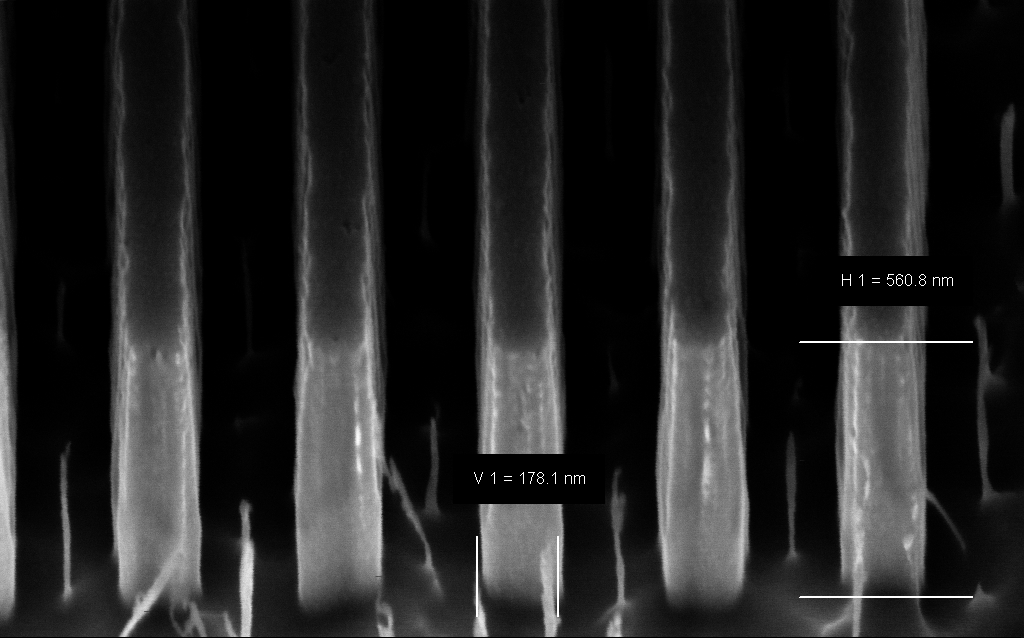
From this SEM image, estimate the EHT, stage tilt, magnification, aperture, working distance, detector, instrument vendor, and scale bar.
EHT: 3 kV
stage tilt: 45°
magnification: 166.97 K X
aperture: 30 µm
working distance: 5 mm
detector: InLens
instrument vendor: Zeiss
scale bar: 200 nm